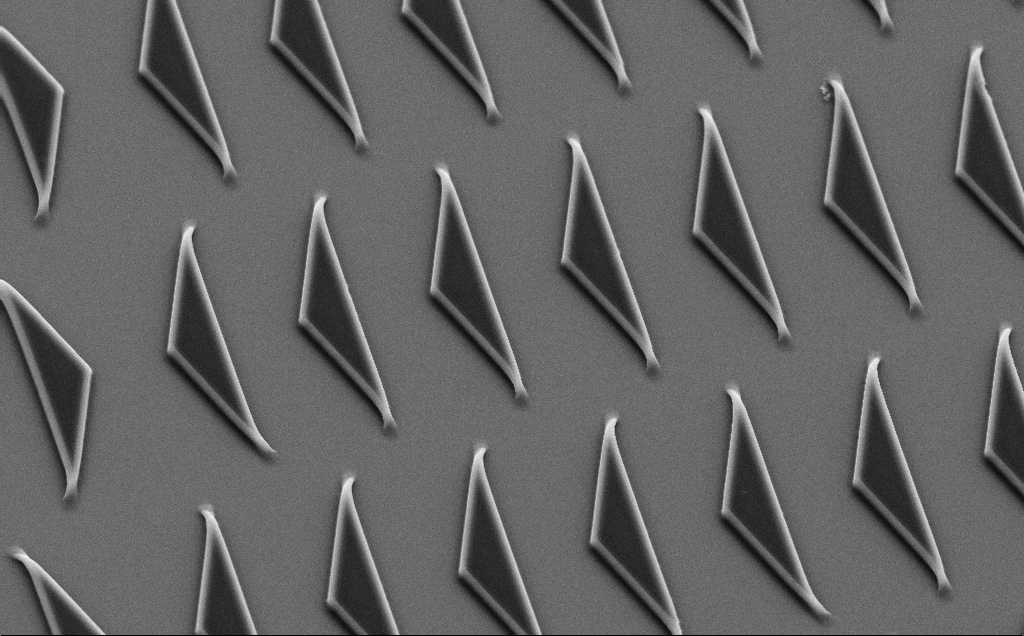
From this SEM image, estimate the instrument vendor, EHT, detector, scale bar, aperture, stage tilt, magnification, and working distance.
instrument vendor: Zeiss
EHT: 10 kV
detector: SE2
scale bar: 10000 nm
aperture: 30 µm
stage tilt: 35°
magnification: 2.96 K X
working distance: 8 mm